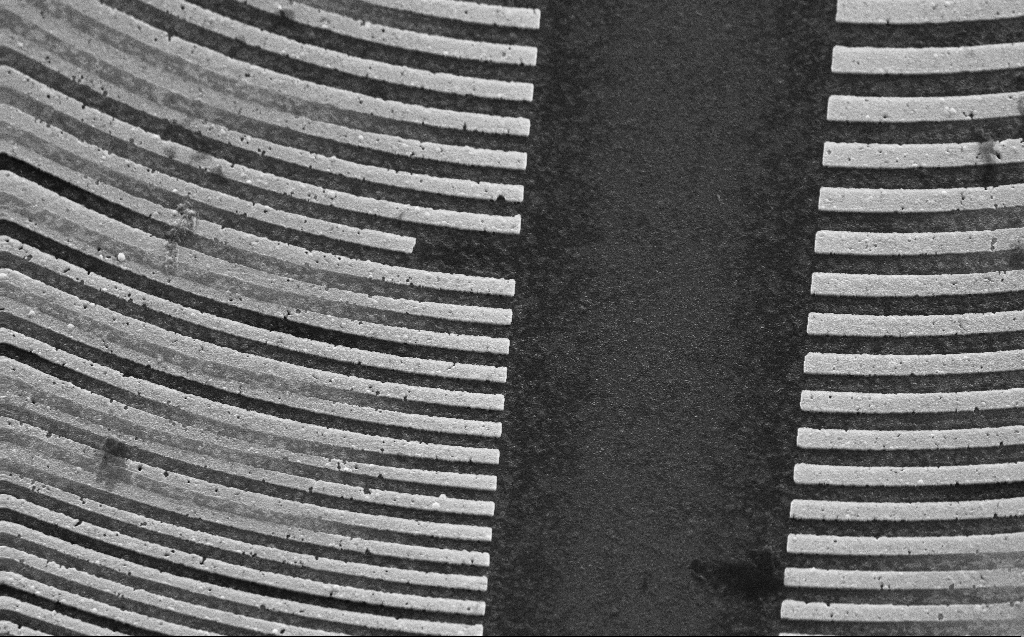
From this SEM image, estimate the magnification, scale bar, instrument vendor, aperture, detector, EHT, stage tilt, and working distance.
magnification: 11.03 K X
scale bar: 2000 nm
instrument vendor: Zeiss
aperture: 30 µm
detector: SE2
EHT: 30 kV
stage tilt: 45°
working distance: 5 mm